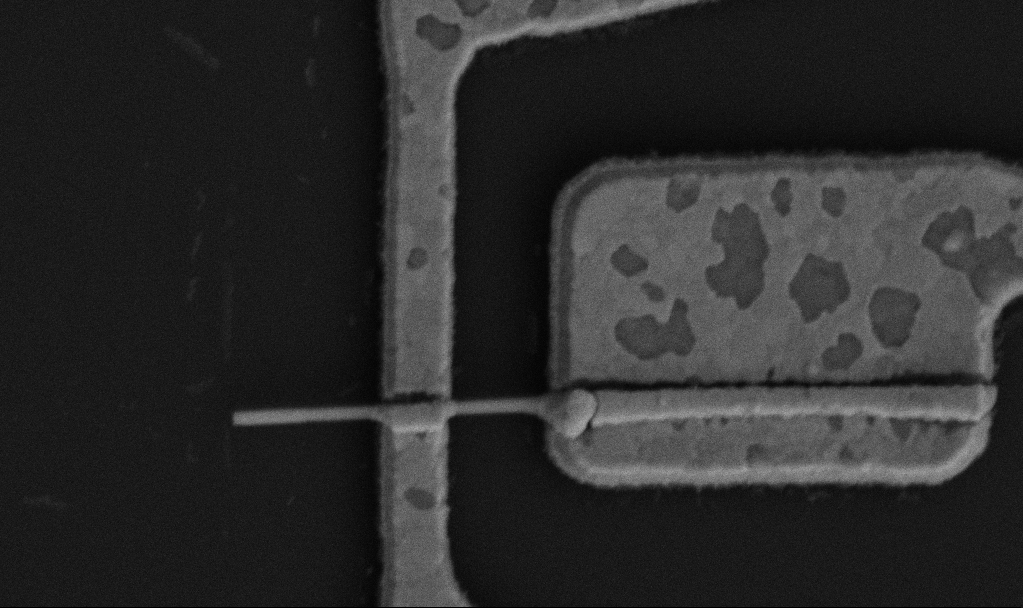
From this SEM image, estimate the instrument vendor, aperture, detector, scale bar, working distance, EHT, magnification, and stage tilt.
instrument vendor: Zeiss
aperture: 30 µm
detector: SE2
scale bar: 1000 nm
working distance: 10.4 mm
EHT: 5 kV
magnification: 50 K X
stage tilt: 0°